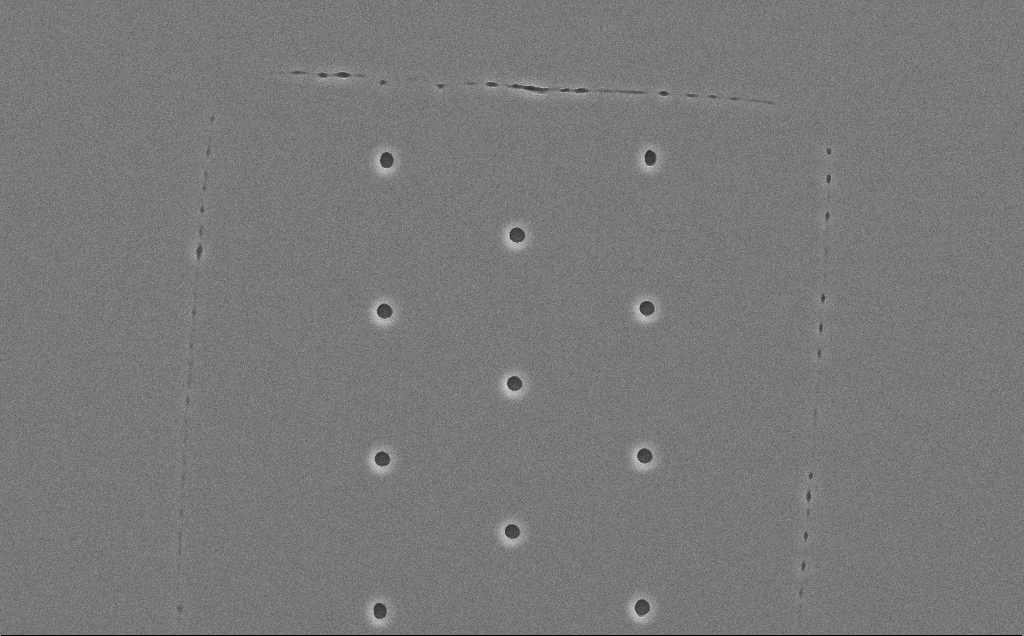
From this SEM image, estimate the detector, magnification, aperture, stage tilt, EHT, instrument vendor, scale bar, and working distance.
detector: SE2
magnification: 2.71 K X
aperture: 30 µm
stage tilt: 0°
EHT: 10 kV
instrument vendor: Zeiss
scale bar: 10000 nm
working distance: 12 mm